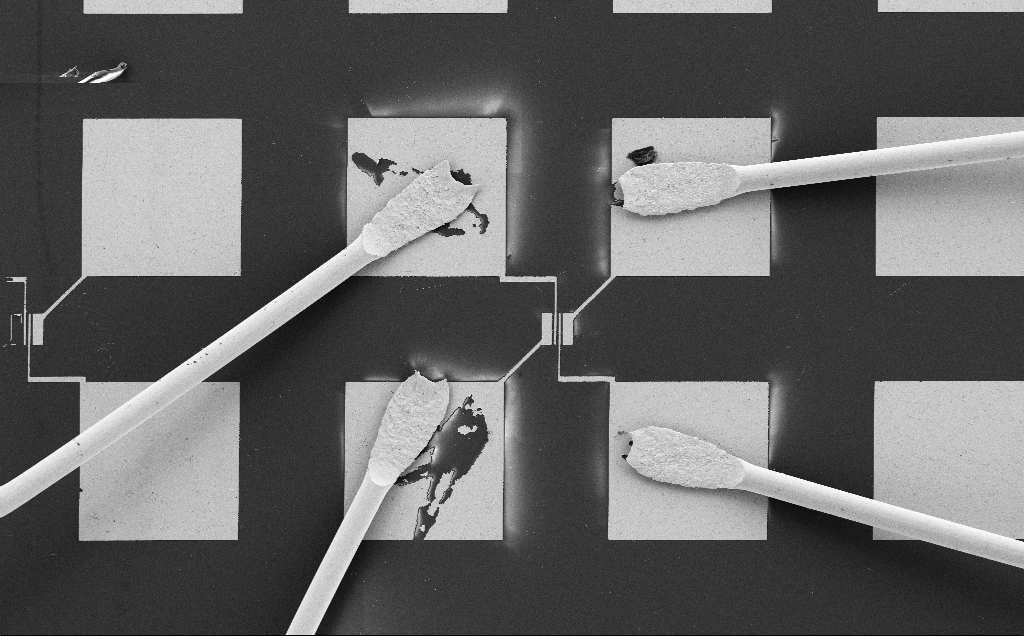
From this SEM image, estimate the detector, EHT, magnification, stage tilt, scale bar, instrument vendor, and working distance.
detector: SE2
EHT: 5 kV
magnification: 0.391 K X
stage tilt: -0.7°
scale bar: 100000 nm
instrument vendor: Zeiss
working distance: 8 mm